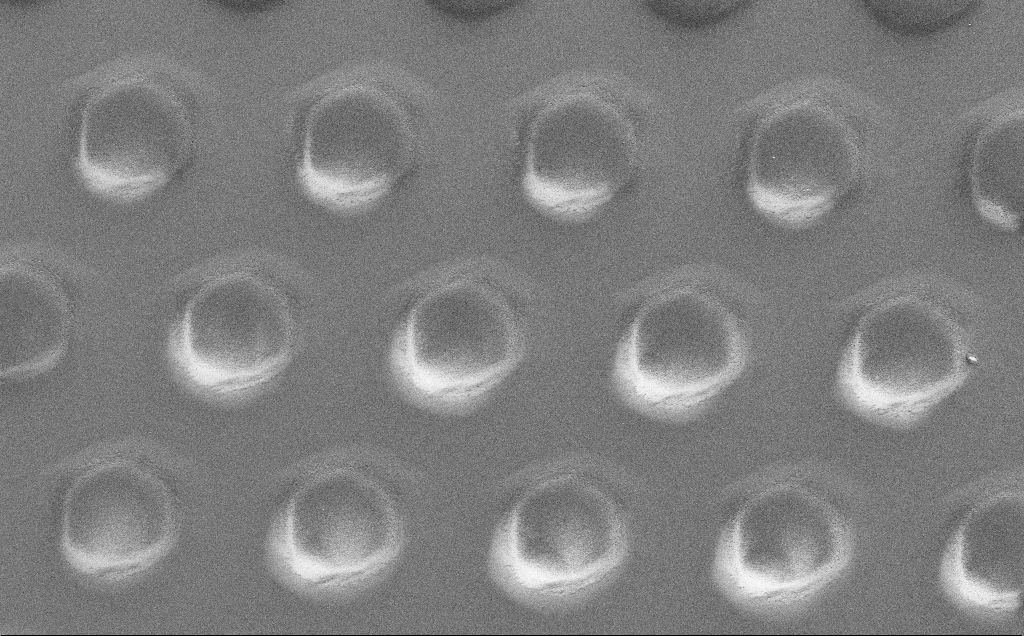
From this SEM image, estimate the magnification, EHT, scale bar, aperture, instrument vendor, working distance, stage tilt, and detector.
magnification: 4.13 K X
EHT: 1.5 kV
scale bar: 10000 nm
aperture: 30 µm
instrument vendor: Zeiss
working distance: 5 mm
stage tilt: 0°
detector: SE2